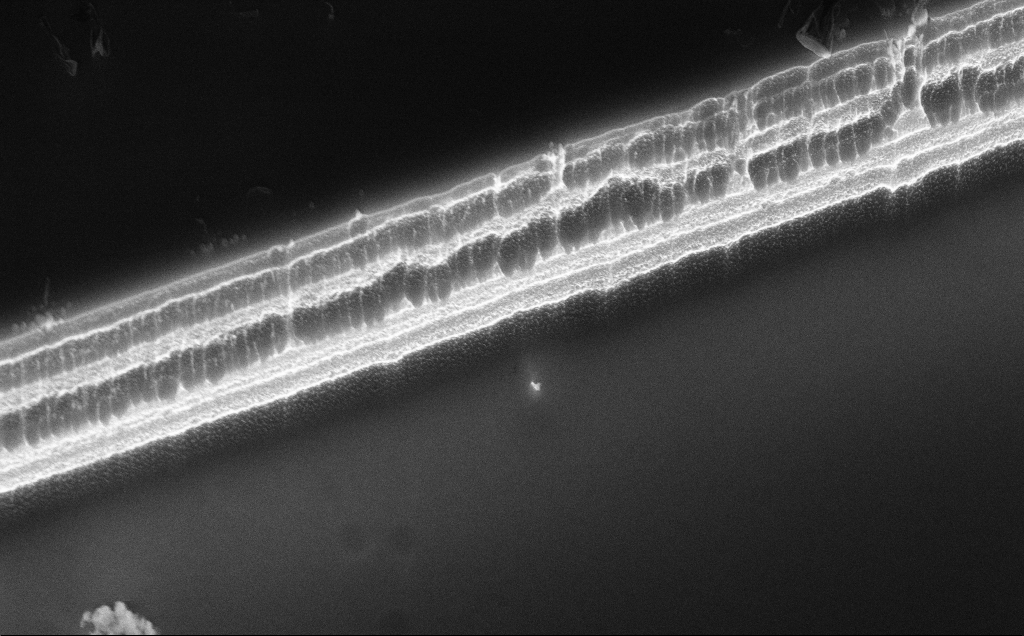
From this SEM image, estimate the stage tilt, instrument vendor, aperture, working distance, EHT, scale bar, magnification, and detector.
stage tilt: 50°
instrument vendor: Zeiss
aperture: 30 µm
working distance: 11 mm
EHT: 10 kV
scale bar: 1000 nm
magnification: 16.15 K X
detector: InLens